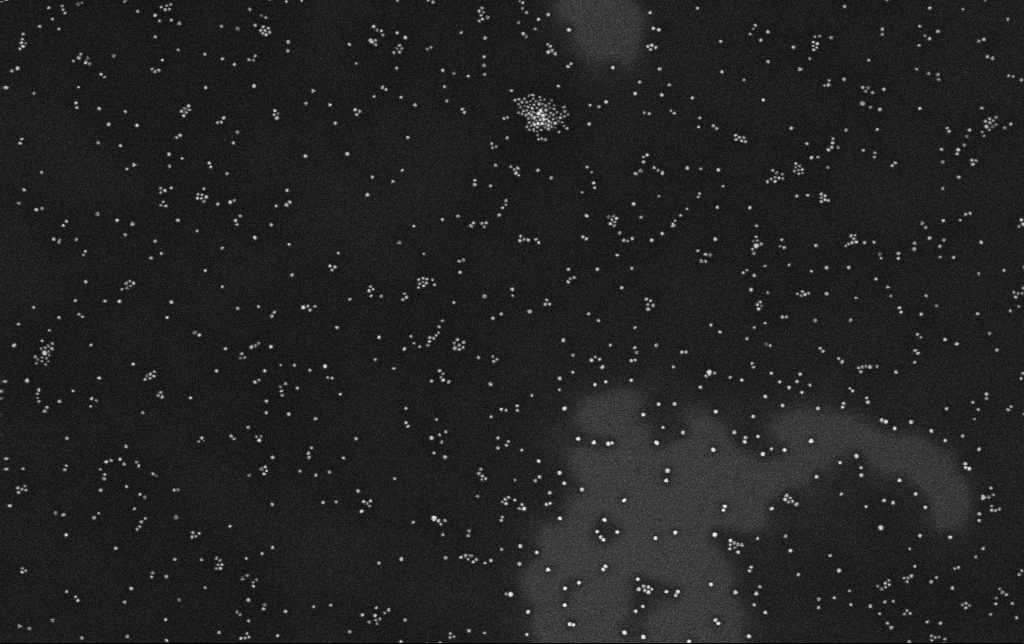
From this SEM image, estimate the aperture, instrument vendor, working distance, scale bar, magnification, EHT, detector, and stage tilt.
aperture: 30 µm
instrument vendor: Zeiss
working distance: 3.3 mm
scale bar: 200 nm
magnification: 100 K X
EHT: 10 kV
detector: InLens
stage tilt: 0°